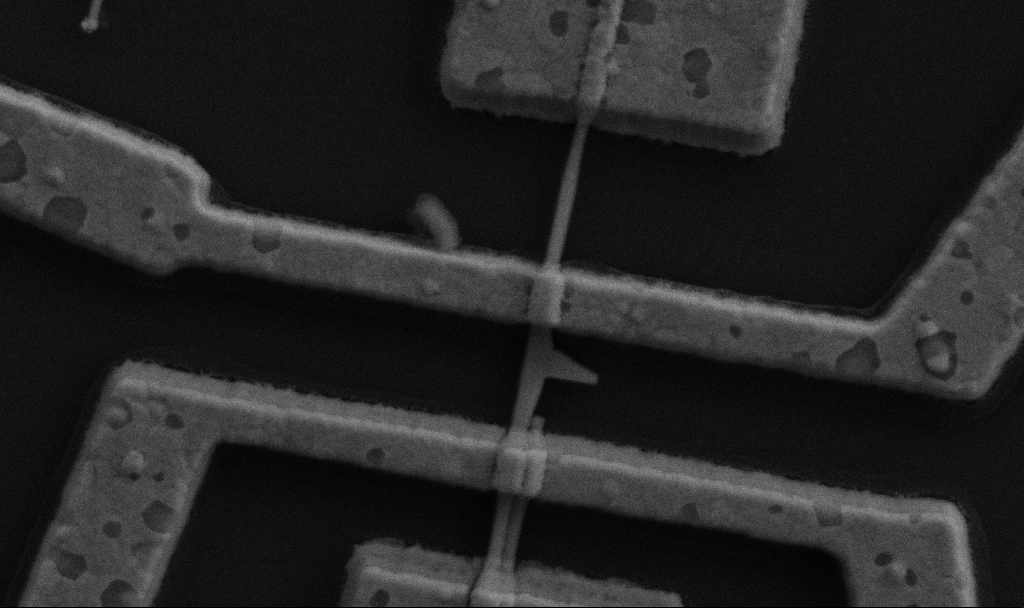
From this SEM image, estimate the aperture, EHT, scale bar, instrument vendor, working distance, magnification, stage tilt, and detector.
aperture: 30 µm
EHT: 5 kV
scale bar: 1000 nm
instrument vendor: Zeiss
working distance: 9.7 mm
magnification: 60 K X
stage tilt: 0°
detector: SE2